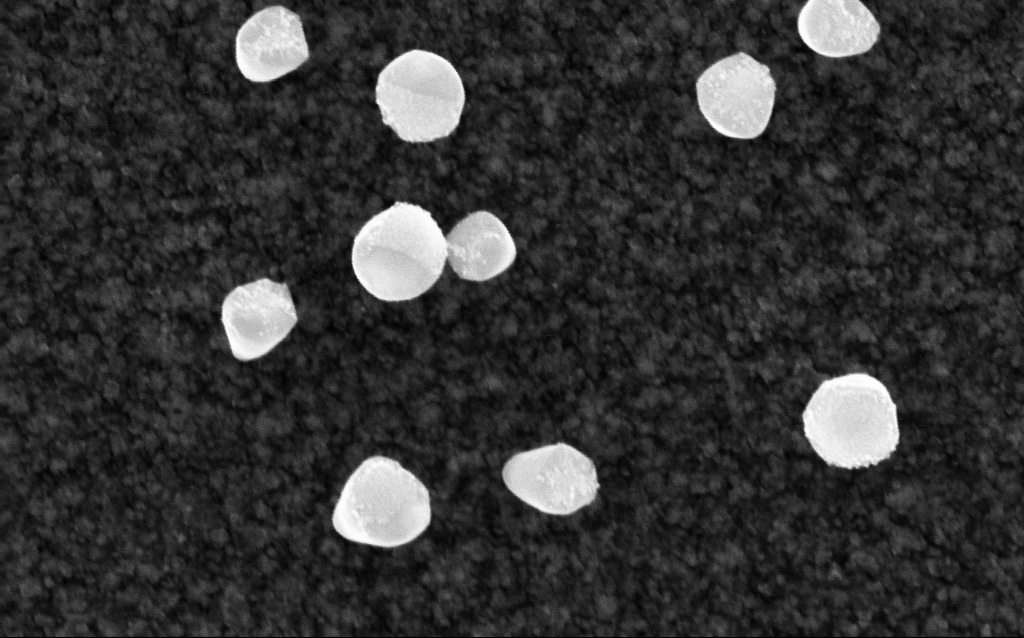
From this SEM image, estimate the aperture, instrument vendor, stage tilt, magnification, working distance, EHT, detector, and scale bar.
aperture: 30 µm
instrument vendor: Zeiss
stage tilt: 0°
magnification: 200 K X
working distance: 2.8 mm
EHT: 5 kV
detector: InLens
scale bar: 100 nm